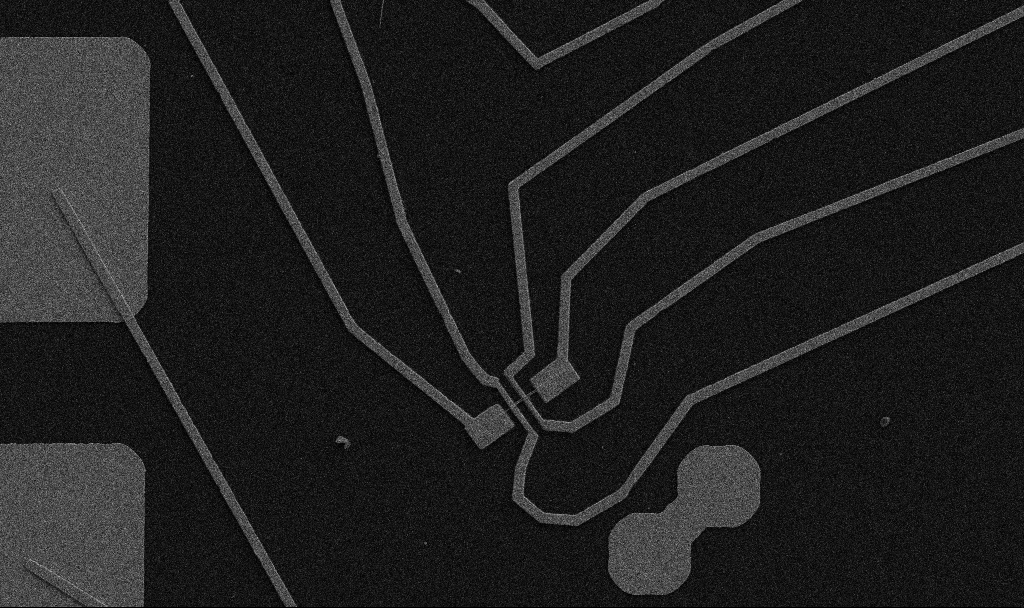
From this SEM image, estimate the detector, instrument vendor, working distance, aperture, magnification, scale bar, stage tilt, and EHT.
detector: SE2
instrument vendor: Zeiss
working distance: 10.7 mm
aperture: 30 µm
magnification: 5 K X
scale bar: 10000 nm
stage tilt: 0°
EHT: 5 kV